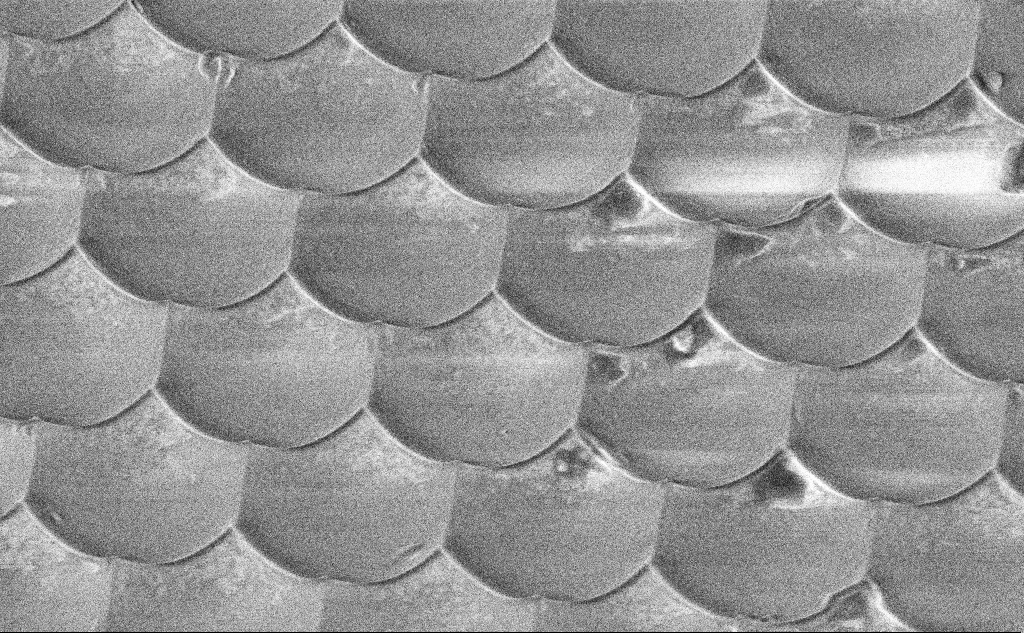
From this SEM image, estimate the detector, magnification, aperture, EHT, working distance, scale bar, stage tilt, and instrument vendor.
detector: InLens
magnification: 25.58 K X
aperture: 30 µm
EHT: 3 kV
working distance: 10.7 mm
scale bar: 2000 nm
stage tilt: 45°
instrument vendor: Zeiss